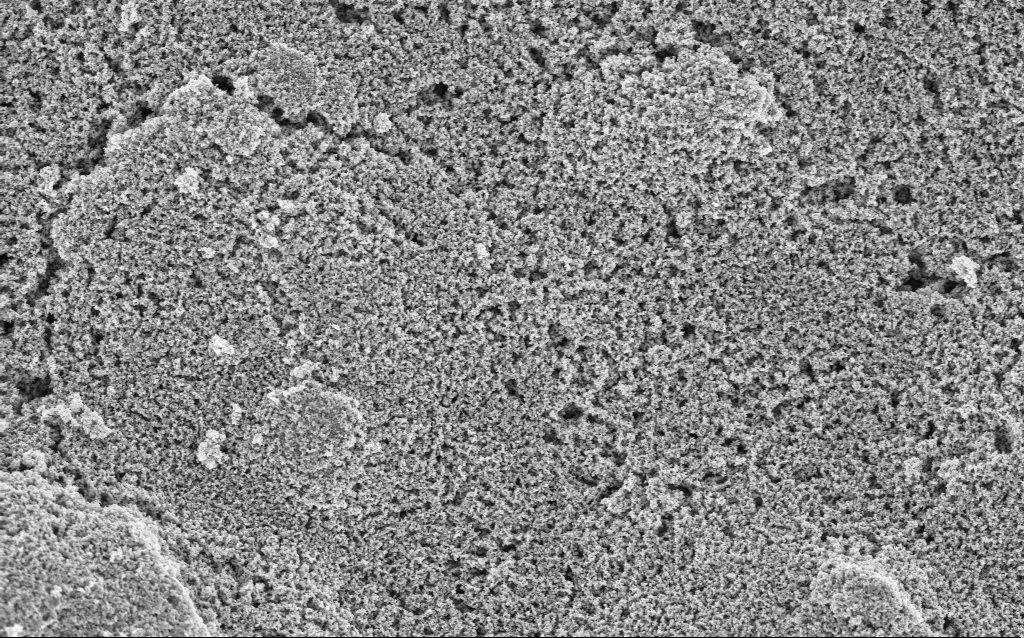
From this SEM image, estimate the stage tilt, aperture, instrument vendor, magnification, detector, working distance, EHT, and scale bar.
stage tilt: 0°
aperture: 30 µm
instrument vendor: Zeiss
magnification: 23.9 K X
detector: InLens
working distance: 7.6 mm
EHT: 3 kV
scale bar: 1000 nm